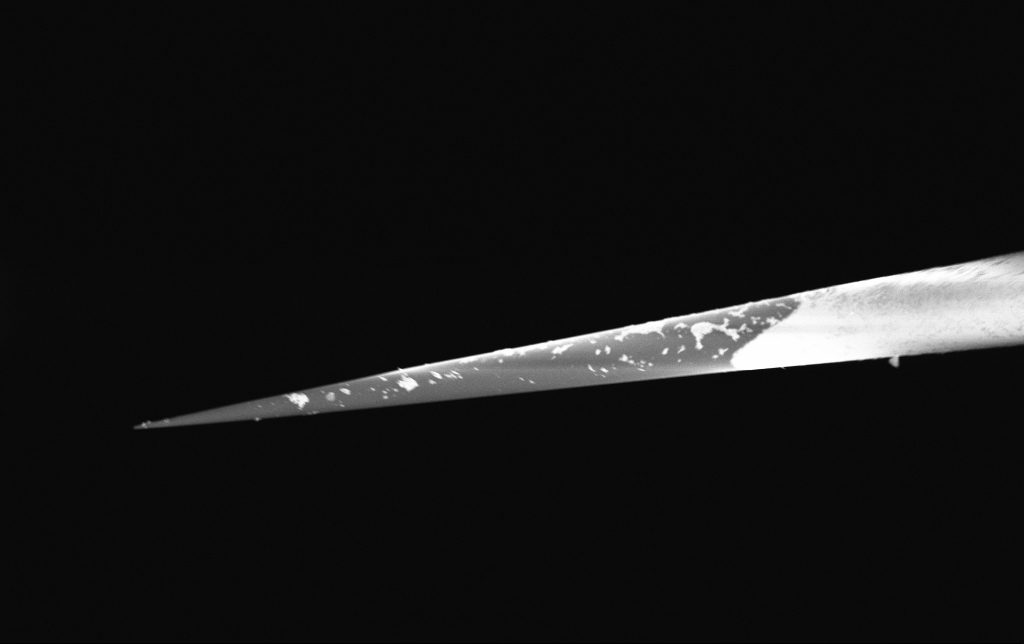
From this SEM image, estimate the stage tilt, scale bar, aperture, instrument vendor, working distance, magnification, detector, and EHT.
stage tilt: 0°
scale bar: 2000 nm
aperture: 30 µm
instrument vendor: Zeiss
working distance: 6.6 mm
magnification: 7.5 K X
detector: InLens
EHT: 2 kV